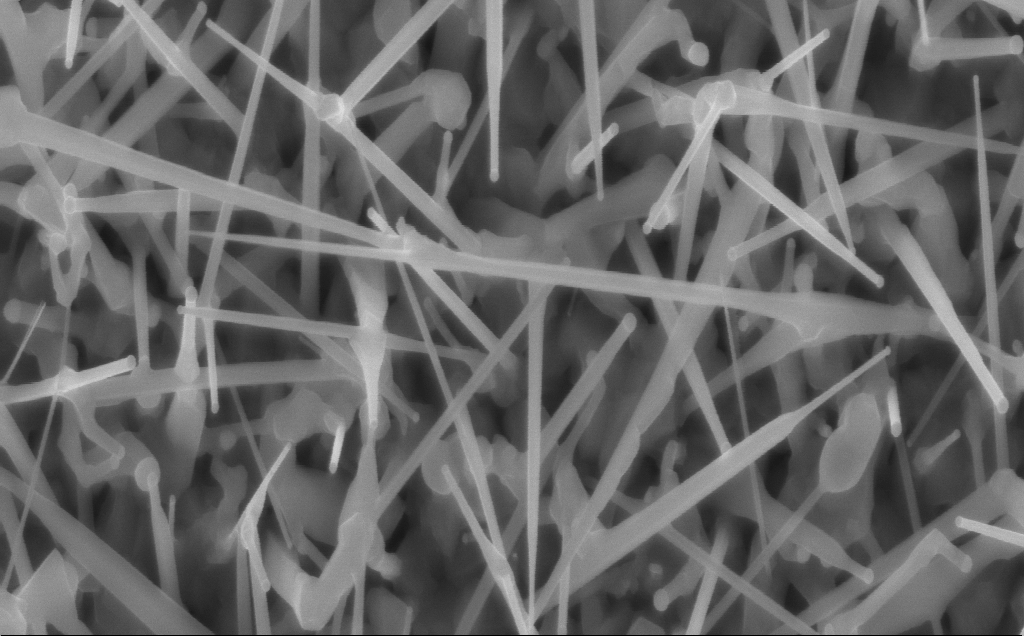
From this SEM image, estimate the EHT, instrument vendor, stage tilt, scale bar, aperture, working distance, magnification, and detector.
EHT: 10 kV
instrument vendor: Zeiss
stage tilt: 0°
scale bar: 1000 nm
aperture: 30 µm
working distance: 4 mm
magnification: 56.78 K X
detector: InLens